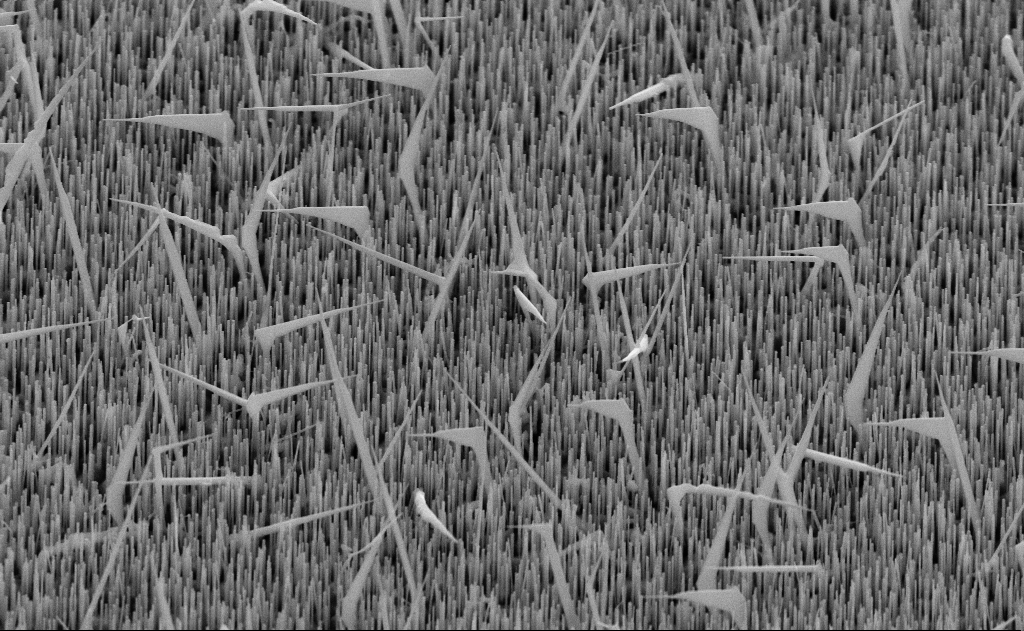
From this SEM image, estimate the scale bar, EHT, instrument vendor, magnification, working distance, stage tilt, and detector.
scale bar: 1000 nm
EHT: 10 kV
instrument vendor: Zeiss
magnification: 20 K X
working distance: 11 mm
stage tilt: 45°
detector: SE2